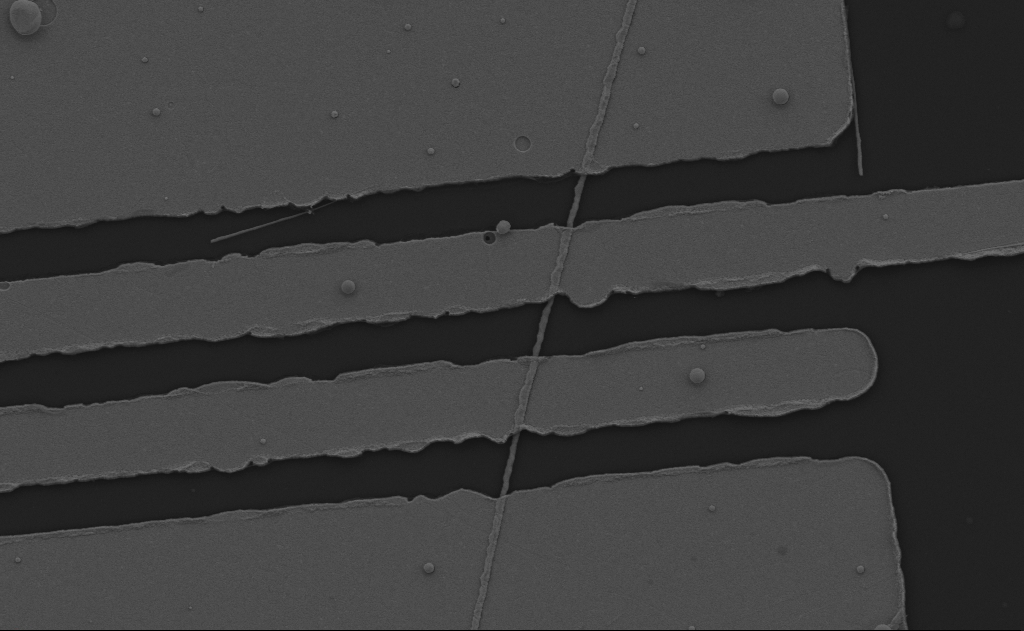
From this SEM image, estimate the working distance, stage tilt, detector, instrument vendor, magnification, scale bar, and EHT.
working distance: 13 mm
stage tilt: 0°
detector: SE2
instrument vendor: Zeiss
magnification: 12.08 K X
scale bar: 2000 nm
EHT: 5 kV